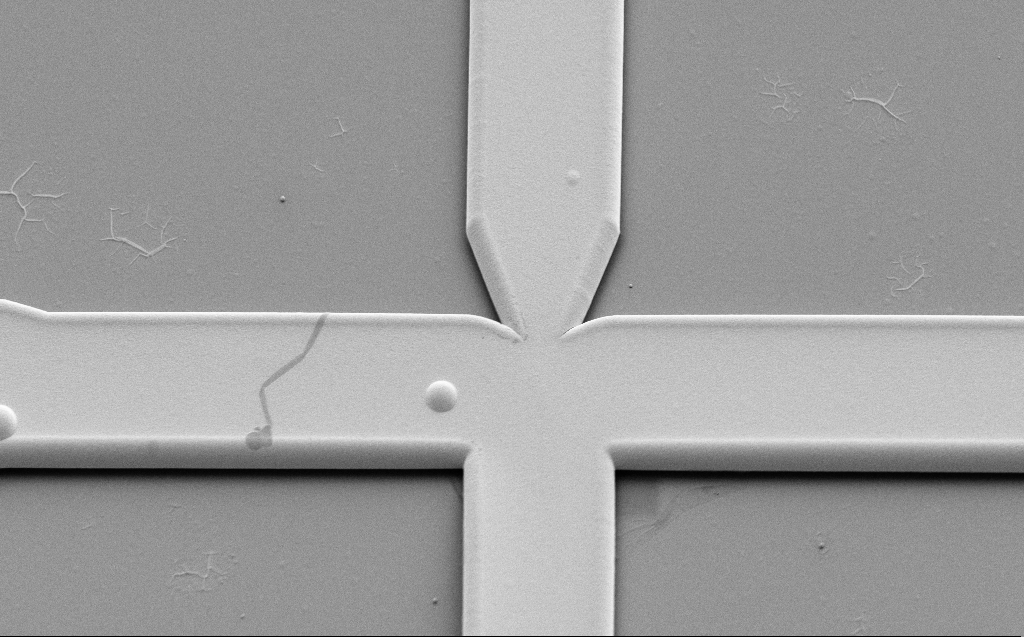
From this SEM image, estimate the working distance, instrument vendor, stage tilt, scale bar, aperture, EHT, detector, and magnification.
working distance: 9 mm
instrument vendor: Zeiss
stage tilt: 45°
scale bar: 10000 nm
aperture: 30 µm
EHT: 5 kV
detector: SE2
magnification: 4.53 K X